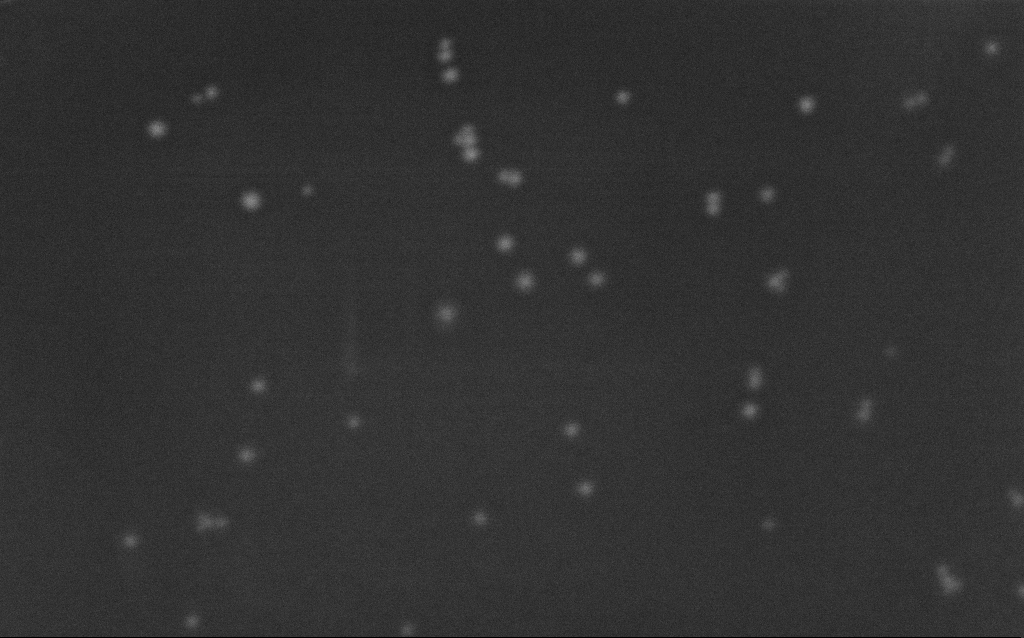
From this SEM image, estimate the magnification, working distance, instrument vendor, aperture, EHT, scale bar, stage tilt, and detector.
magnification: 320.93 K X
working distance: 2.7 mm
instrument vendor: Zeiss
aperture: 30 µm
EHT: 8 kV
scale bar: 100 nm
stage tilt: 0°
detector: InLens